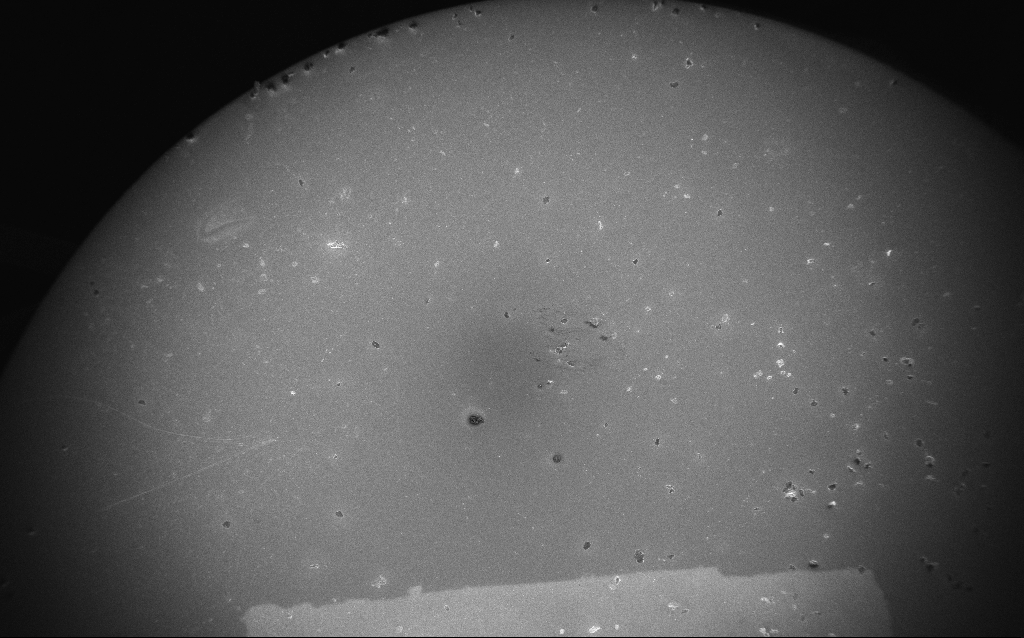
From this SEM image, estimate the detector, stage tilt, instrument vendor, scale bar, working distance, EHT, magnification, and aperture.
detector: InLens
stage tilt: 0°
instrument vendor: Zeiss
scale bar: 200000 nm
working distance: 5 mm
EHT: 5 kV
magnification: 0.089 K X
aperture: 30 µm